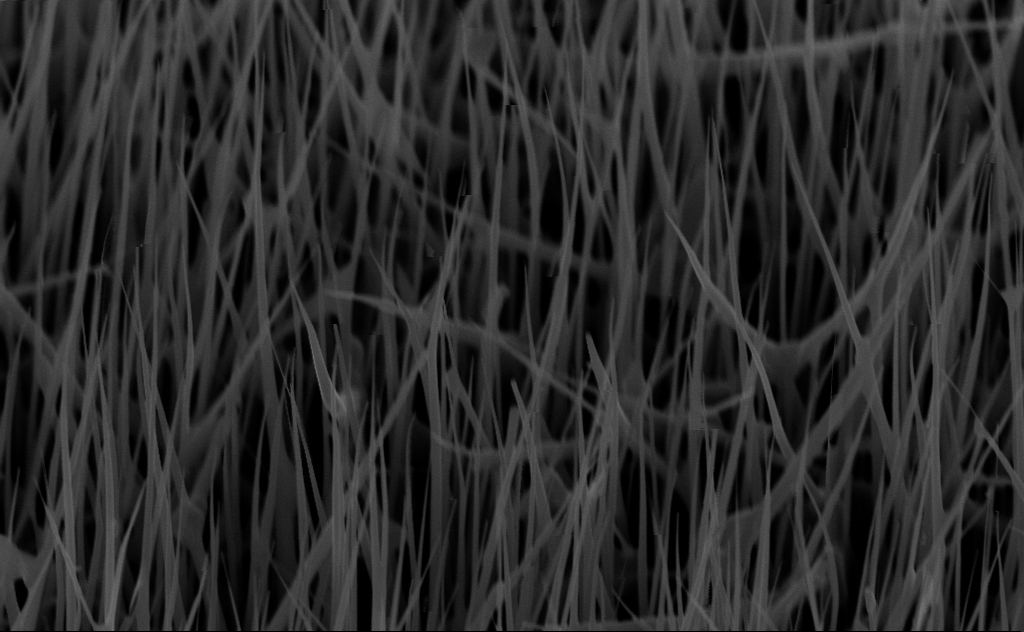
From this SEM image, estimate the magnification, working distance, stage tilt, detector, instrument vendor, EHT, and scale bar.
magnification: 40 K X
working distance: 6 mm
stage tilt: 45°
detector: InLens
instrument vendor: Zeiss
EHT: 10 kV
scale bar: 1000 nm